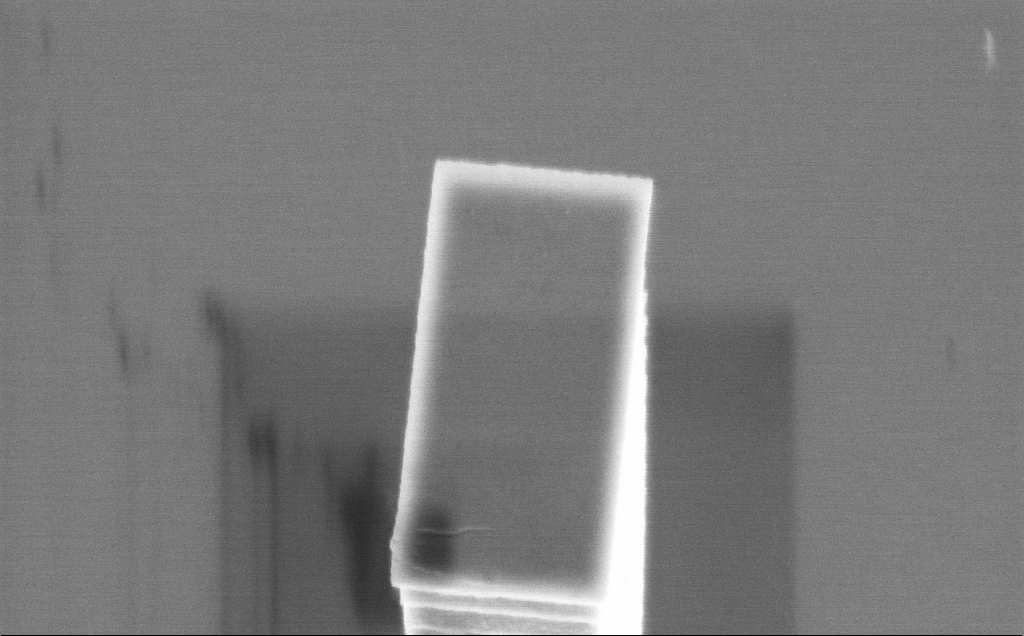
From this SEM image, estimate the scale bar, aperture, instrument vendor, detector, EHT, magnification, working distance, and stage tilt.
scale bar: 1000 nm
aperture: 30 µm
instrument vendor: Zeiss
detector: InLens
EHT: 5 kV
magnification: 28.52 K X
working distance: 3 mm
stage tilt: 36.8°